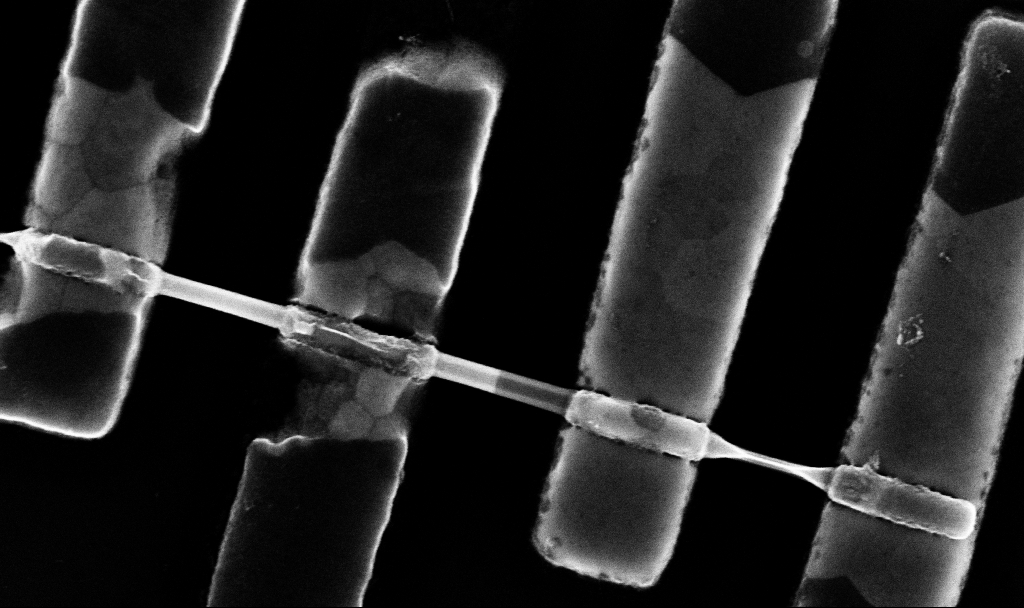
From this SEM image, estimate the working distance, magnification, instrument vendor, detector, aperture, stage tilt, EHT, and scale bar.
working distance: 6.8 mm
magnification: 90.76 K X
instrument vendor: Zeiss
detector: InLens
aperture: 30 µm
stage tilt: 0°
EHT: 10 kV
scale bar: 200 nm